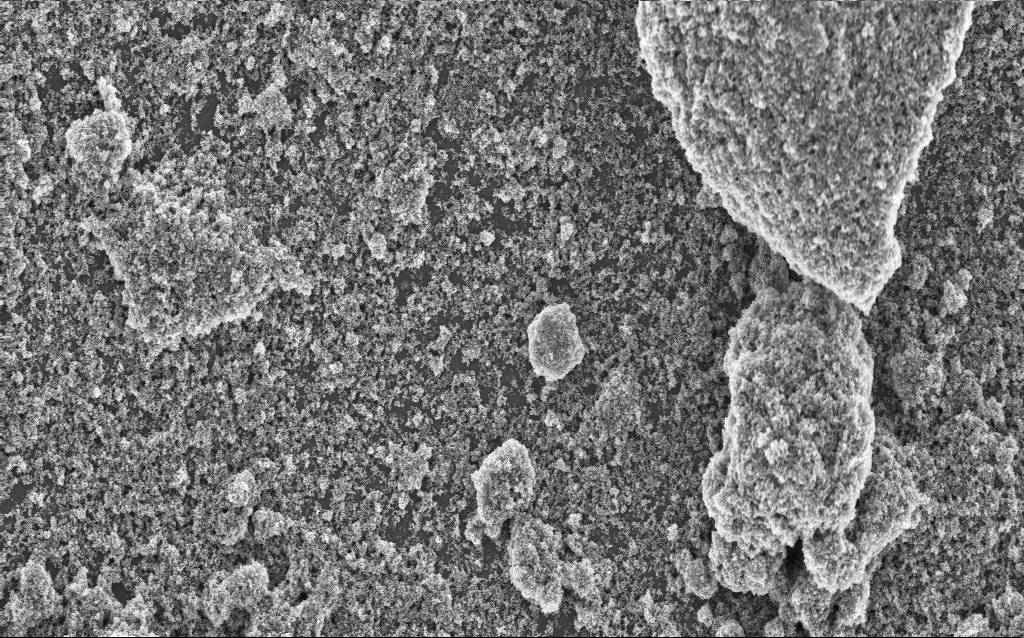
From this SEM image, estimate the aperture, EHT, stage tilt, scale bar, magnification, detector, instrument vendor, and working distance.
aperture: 30 µm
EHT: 5 kV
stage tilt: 0°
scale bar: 2000 nm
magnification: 9.04 K X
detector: InLens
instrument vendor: Zeiss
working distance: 4.7 mm